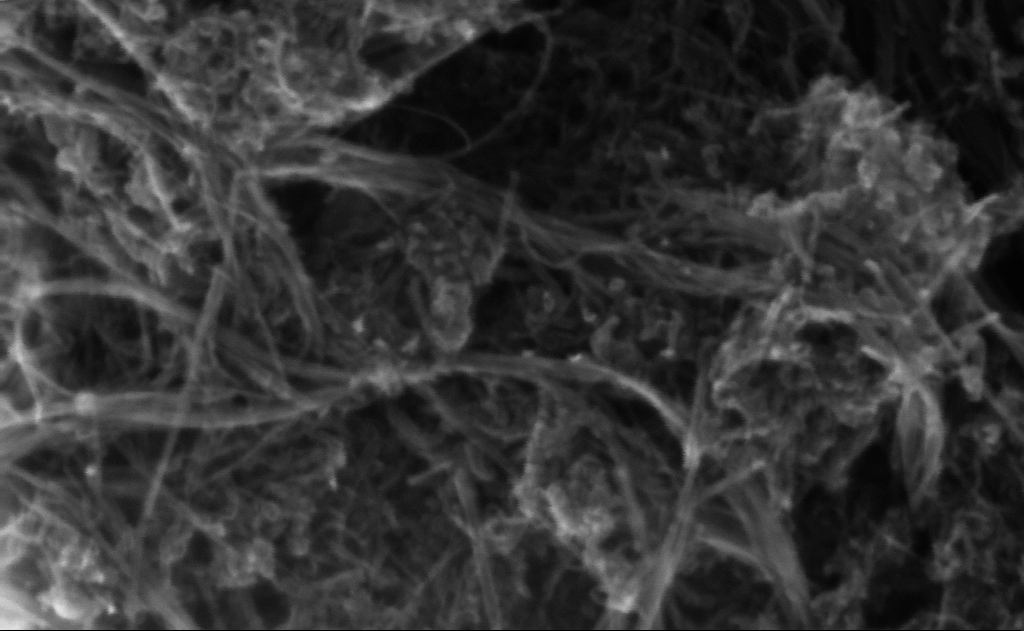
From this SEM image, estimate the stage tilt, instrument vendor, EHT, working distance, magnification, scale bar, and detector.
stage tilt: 0°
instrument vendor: Zeiss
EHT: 10 kV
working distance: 3 mm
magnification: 553.46 K X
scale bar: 100 nm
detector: InLens